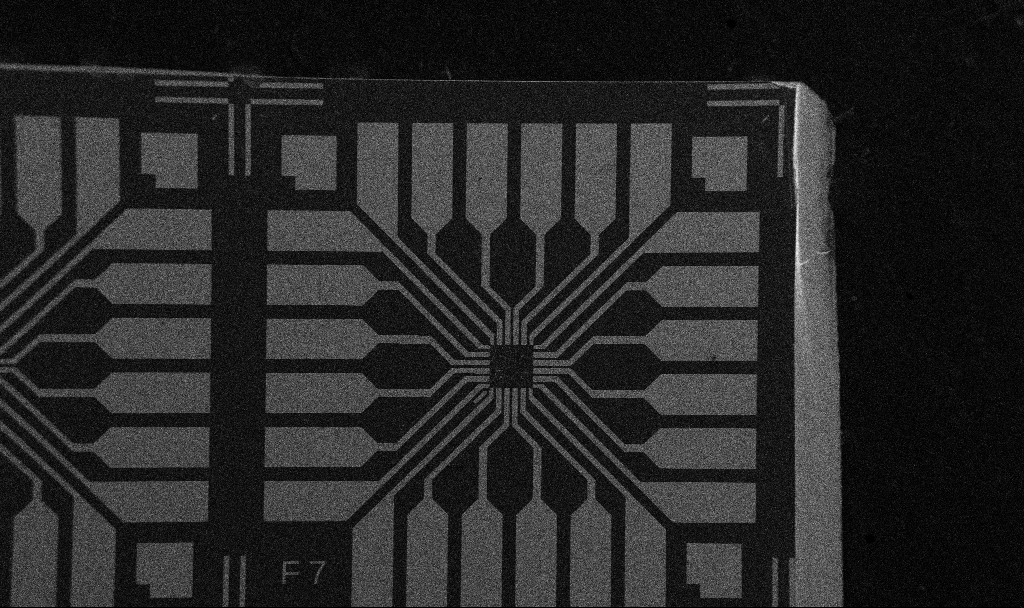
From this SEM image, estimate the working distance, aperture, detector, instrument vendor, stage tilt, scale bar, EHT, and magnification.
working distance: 10.7 mm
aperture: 30 µm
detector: SE2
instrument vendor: Zeiss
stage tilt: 0°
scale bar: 200000 nm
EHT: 5 kV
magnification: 0.1 K X